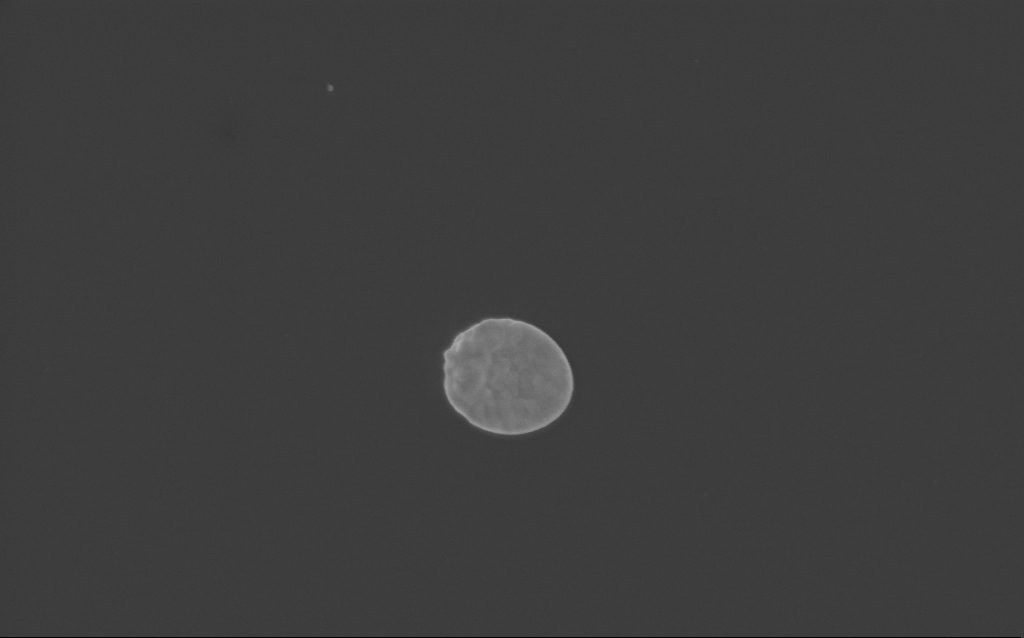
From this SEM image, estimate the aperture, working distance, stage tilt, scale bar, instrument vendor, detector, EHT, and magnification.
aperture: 30 µm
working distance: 3 mm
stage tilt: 0°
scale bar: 1000 nm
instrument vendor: Zeiss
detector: InLens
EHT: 3 kV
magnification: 51.17 K X